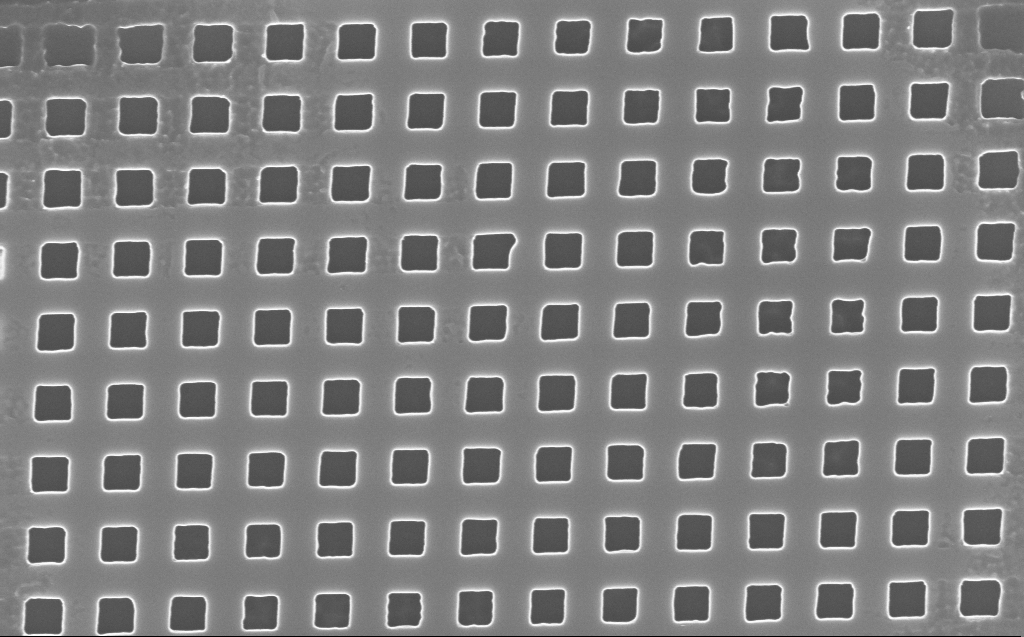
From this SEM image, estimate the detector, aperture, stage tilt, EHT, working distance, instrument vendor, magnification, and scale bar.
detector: InLens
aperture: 30 µm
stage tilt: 0°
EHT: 10 kV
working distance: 4 mm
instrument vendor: Zeiss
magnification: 53.42 K X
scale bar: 1000 nm